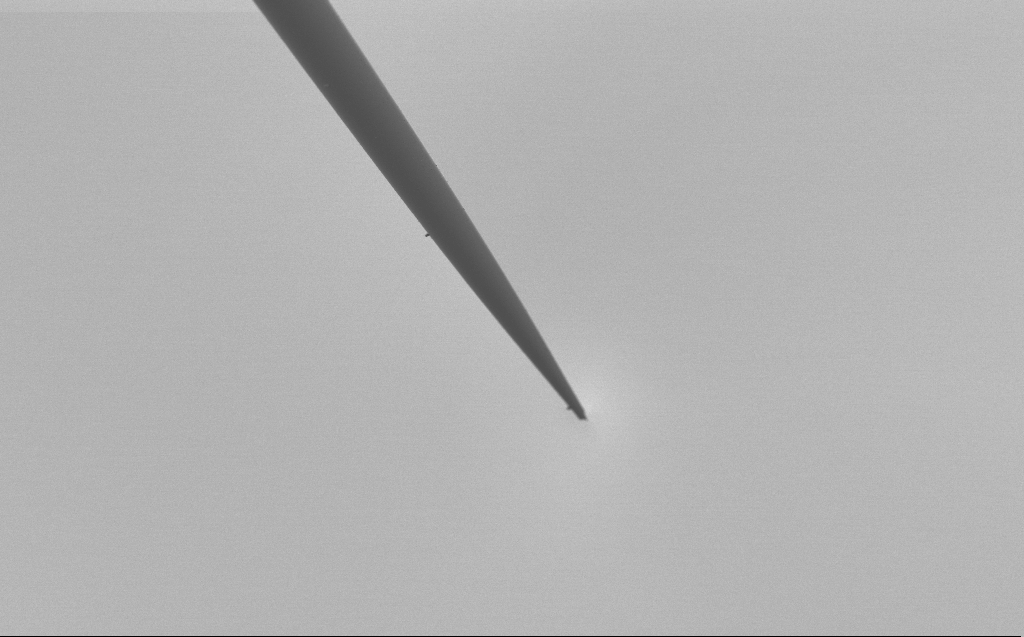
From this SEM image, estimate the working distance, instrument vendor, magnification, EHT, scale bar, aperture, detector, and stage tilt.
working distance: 4 mm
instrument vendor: Zeiss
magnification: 1 K X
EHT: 2 kV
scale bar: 20000 nm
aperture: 30 µm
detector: SE2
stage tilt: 45°